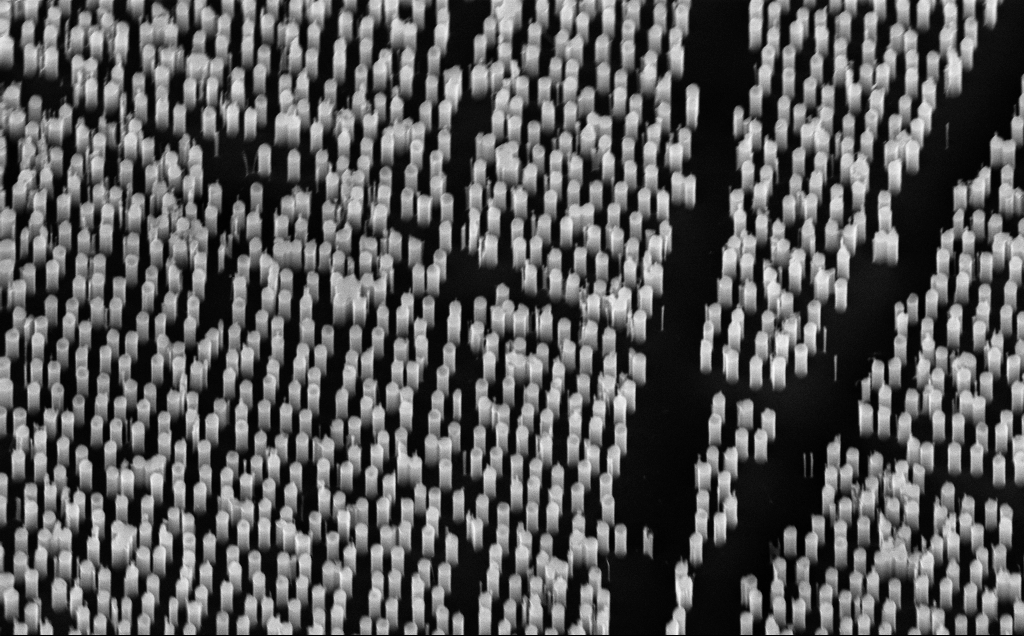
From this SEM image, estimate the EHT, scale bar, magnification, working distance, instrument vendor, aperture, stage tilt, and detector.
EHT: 10 kV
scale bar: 2000 nm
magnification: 21.76 K X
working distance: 6 mm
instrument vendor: Zeiss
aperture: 30 µm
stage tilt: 45°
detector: InLens